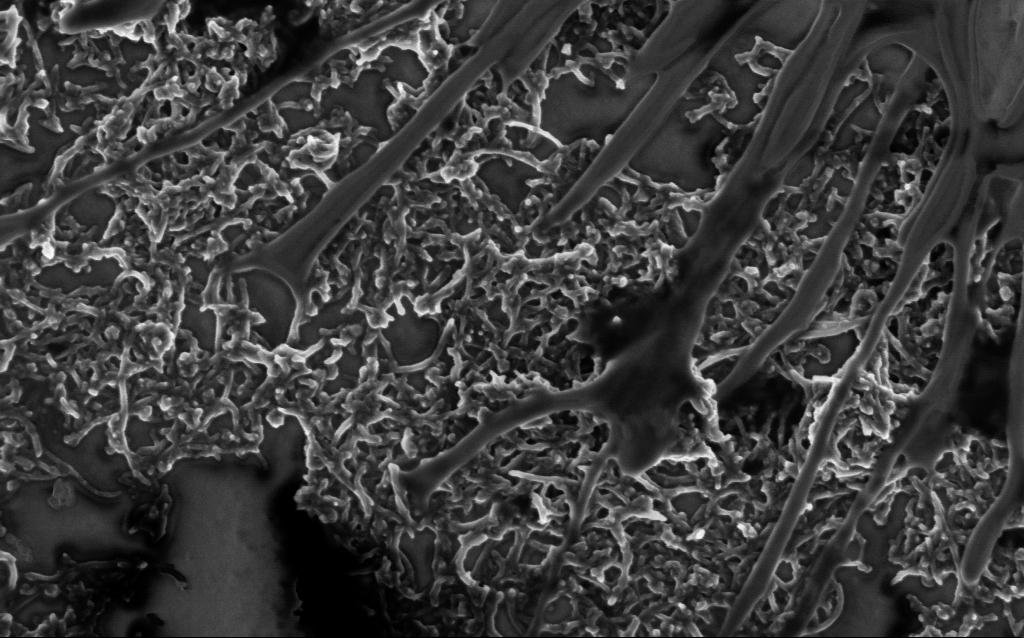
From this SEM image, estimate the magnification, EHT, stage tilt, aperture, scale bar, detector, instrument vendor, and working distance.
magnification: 50 K X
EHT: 2 kV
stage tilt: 0°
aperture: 30 µm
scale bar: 1000 nm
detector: InLens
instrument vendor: Zeiss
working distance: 6.9 mm